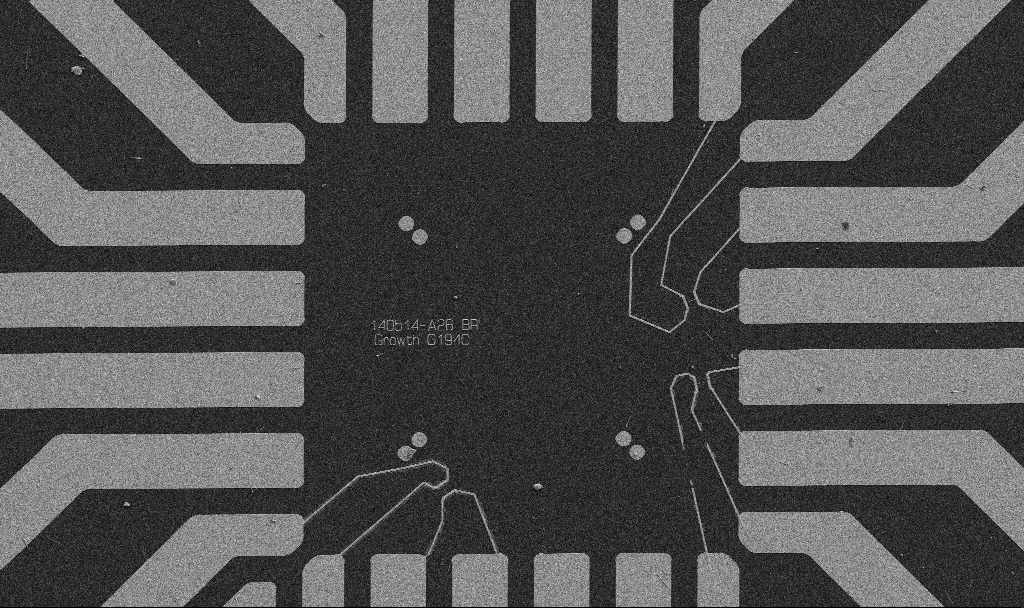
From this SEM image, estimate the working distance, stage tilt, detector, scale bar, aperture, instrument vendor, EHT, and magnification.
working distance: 10.7 mm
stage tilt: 0°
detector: SE2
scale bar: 20000 nm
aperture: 30 µm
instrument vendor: Zeiss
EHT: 5 kV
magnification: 1 K X